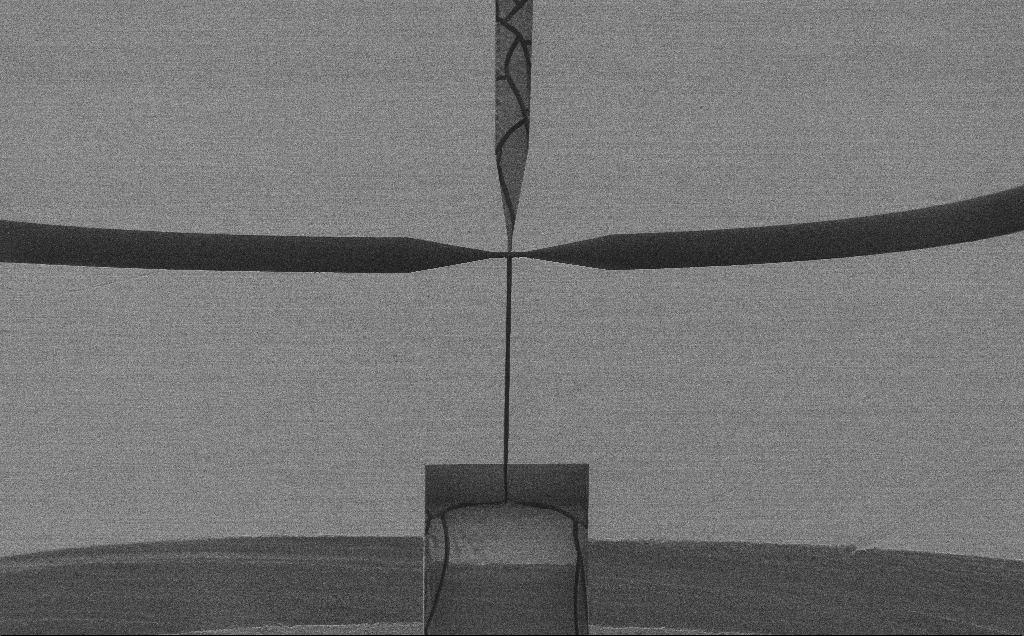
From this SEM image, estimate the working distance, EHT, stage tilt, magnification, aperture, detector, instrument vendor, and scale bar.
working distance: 6 mm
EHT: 1.2 kV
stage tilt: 45°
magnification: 0.151 K X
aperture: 30 µm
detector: SE2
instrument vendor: Zeiss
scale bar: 100000 nm